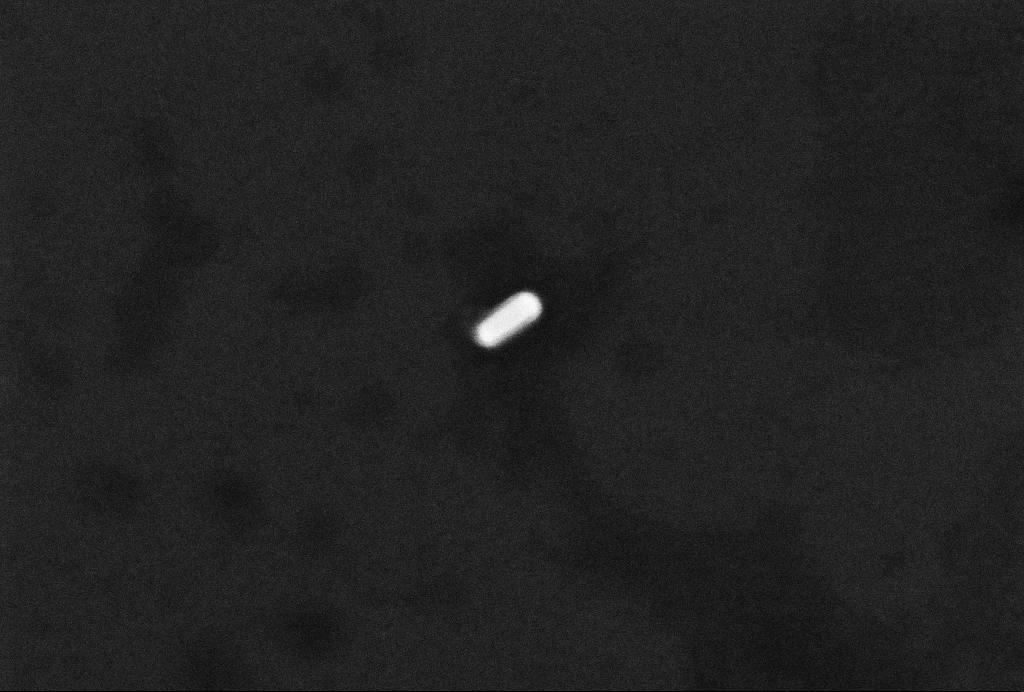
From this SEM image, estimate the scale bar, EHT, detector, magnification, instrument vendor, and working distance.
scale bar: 100 nm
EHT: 10 kV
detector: InLens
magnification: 350 K X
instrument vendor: Zeiss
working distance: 3.2 mm